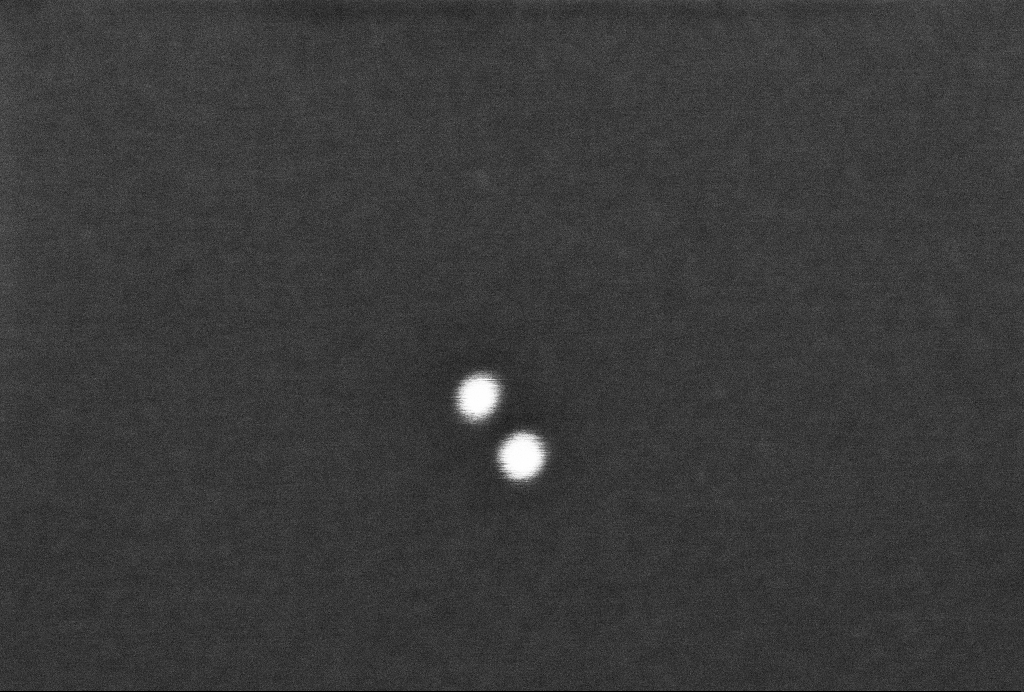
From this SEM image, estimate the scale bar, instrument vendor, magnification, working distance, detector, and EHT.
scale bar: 100 nm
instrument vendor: Zeiss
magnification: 560.02 K X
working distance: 3.3 mm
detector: InLens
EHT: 2 kV